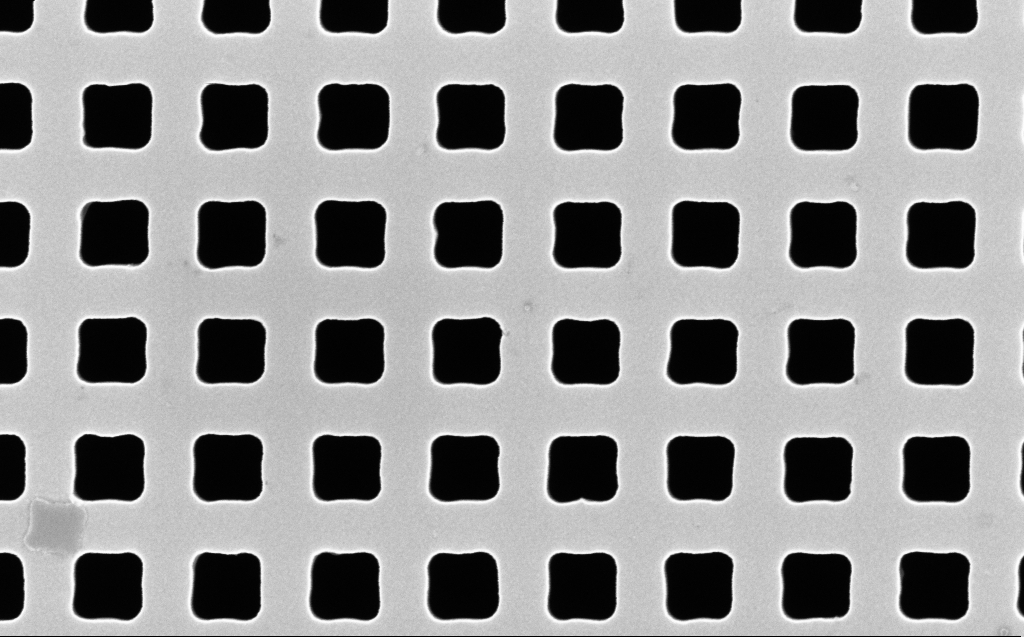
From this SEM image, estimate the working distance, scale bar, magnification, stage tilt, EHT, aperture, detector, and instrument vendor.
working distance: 7 mm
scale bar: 200 nm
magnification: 87.42 K X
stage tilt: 0°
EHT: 10 kV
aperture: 30 µm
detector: InLens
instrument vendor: Zeiss